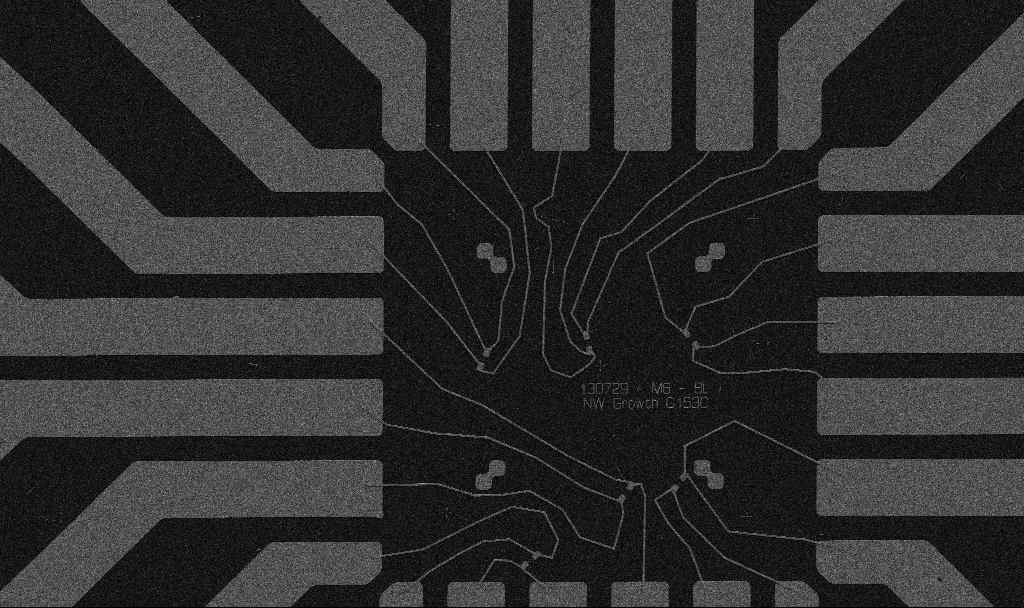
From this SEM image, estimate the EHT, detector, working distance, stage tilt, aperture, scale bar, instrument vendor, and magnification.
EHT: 5 kV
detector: SE2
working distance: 10.7 mm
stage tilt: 0°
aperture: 30 µm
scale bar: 20000 nm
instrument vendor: Zeiss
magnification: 1 K X